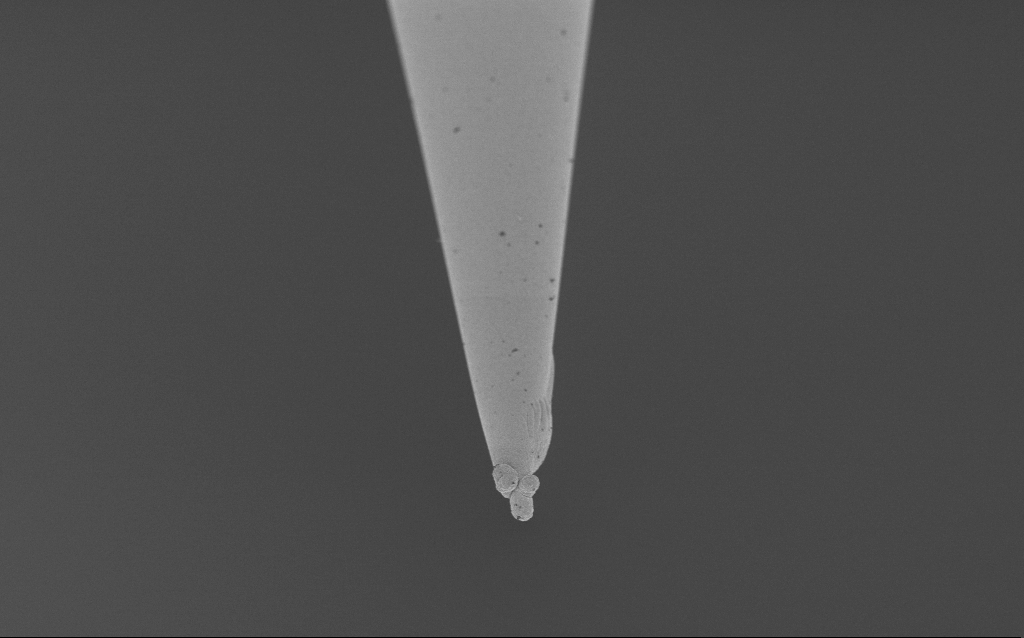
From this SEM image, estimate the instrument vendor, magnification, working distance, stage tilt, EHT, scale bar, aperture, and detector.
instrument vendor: Zeiss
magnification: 5 K X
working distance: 4.9 mm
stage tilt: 45°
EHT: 2 kV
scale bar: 10000 nm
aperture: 30 µm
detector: InLens